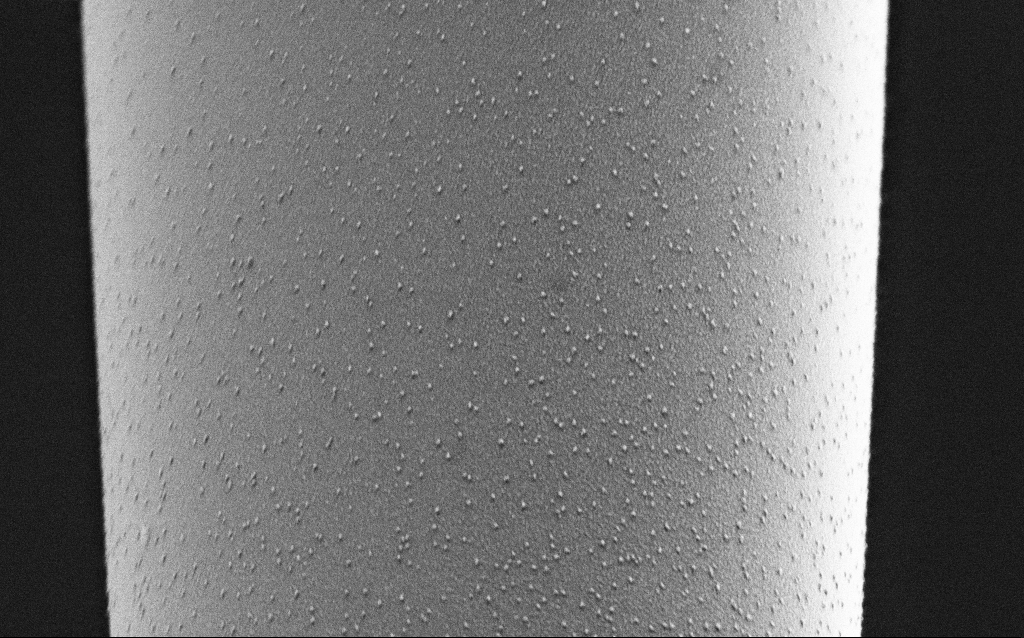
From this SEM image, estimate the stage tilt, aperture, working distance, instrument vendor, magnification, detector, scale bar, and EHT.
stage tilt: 44.9°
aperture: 30 µm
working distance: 6.8 mm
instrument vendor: Zeiss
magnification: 20 K X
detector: SE2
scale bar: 2000 nm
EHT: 2 kV